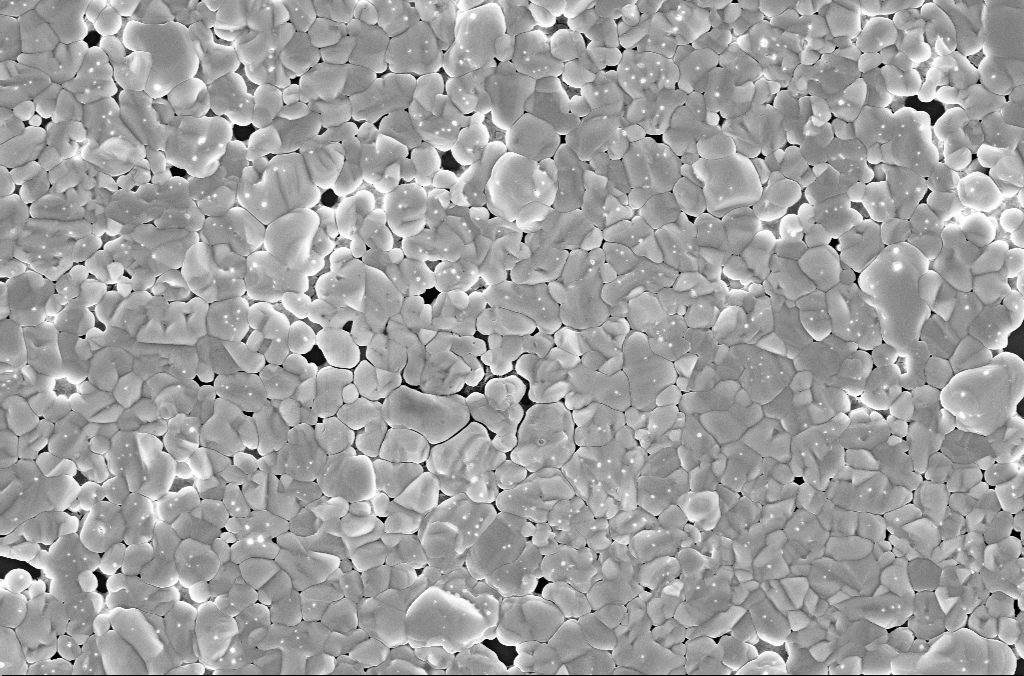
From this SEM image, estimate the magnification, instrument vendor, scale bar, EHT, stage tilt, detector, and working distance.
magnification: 20 K X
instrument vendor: Zeiss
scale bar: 2000 nm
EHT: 5 kV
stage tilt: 0°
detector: InLens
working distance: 3.1 mm